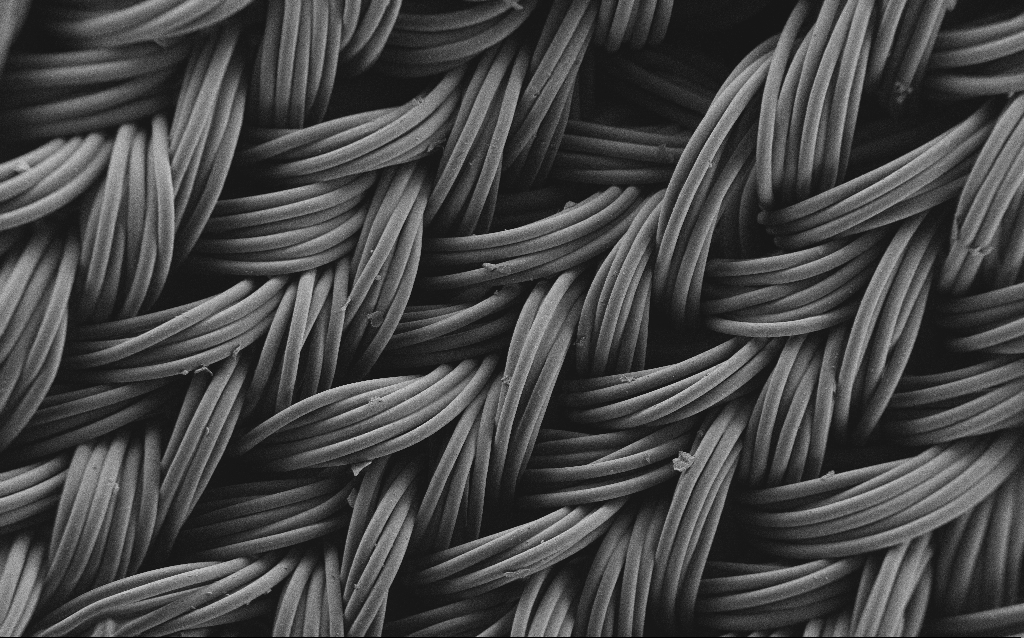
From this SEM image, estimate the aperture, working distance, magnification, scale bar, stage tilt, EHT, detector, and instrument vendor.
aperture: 30 µm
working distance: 4 mm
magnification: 0.225 K X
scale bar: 100000 nm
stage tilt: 0°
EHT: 1 kV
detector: SE2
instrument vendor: Zeiss